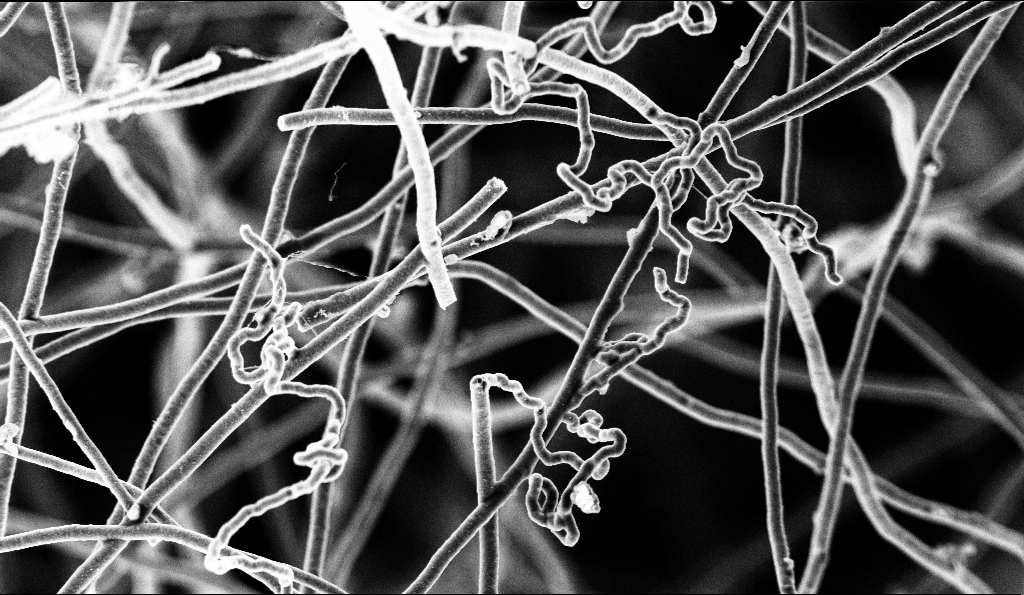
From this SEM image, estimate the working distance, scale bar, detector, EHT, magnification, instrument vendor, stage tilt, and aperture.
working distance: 5.5 mm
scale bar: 2000 nm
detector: InLens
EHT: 3 kV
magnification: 10 K X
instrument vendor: Zeiss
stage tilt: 0°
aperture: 30 µm